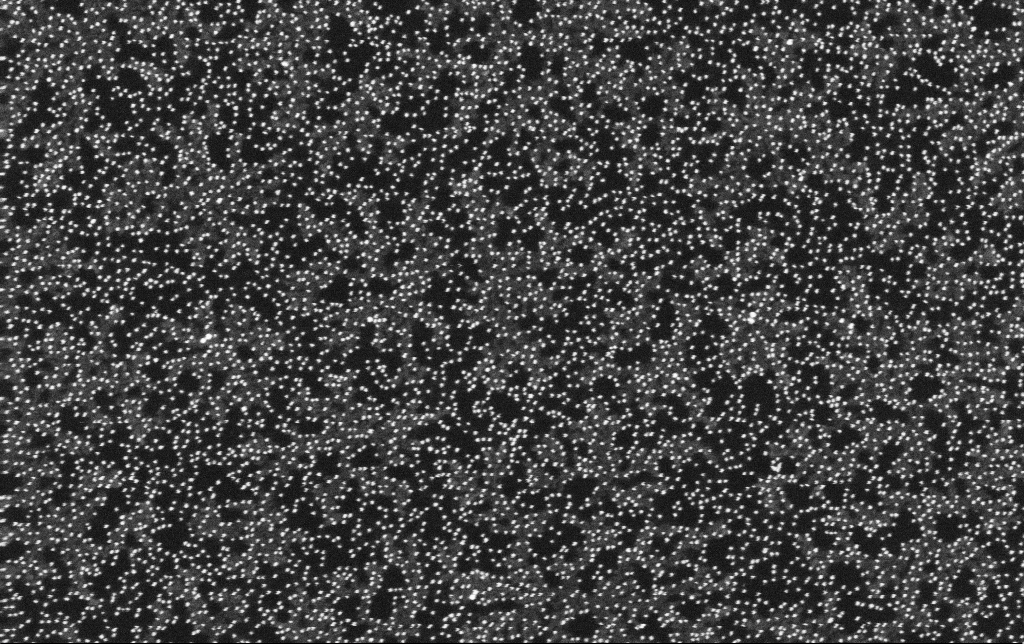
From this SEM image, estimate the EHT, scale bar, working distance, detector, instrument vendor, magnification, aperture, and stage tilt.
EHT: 20 kV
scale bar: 200 nm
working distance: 11.3 mm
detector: SE2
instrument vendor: Zeiss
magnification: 100 K X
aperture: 30 µm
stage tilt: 0°